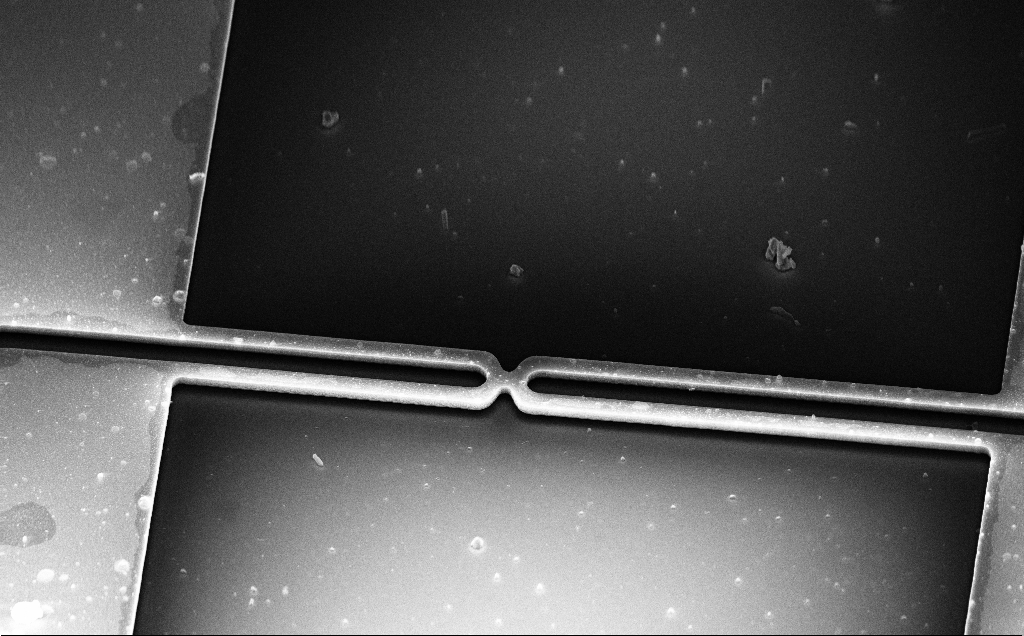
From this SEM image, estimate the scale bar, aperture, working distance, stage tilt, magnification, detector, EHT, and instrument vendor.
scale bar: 20000 nm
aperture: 30 µm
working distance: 5 mm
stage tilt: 58°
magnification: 1.01 K X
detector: InLens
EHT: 15 kV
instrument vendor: Zeiss